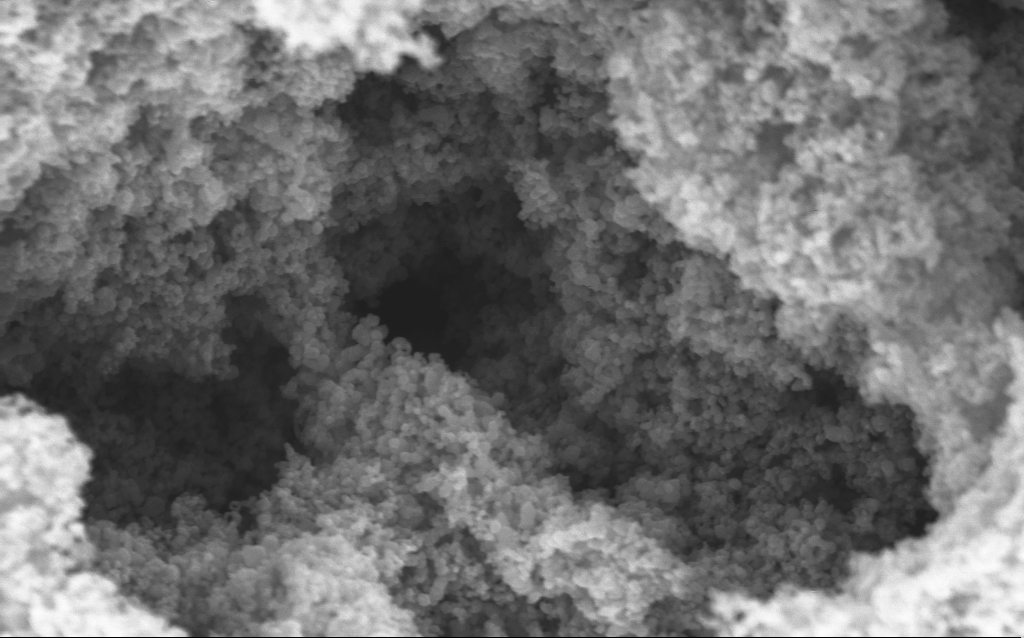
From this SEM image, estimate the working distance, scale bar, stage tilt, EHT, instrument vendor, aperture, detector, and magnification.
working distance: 4.4 mm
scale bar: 200 nm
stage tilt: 0°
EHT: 5 kV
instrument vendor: Zeiss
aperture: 30 µm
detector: InLens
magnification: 114.68 K X